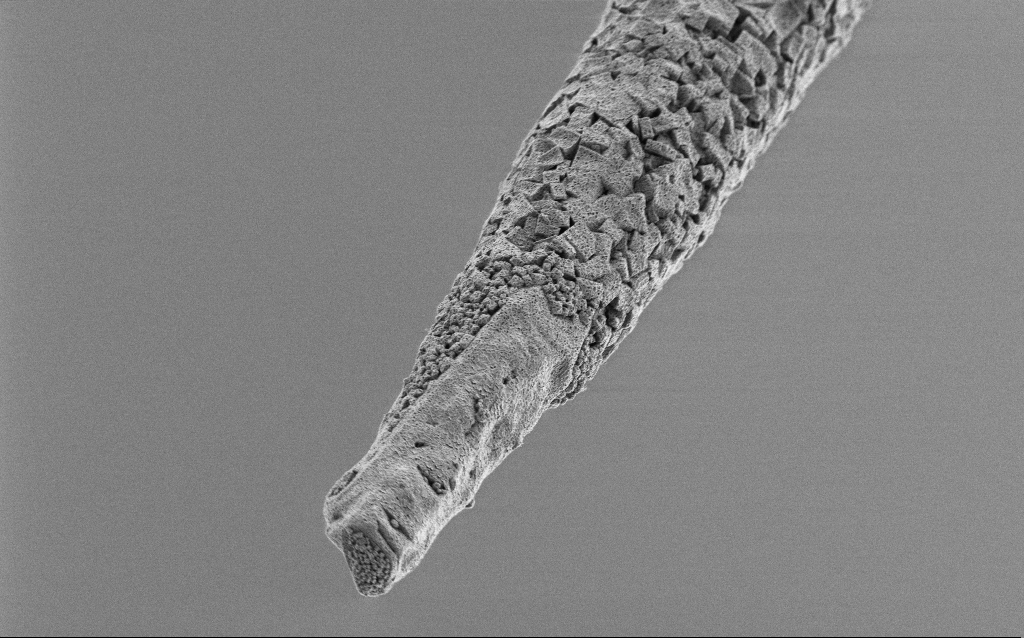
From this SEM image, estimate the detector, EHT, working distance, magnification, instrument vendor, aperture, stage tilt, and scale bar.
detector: SE2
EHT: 1 kV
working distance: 6.8 mm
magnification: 5 K X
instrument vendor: Zeiss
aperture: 30 µm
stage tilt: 45°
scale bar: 10000 nm